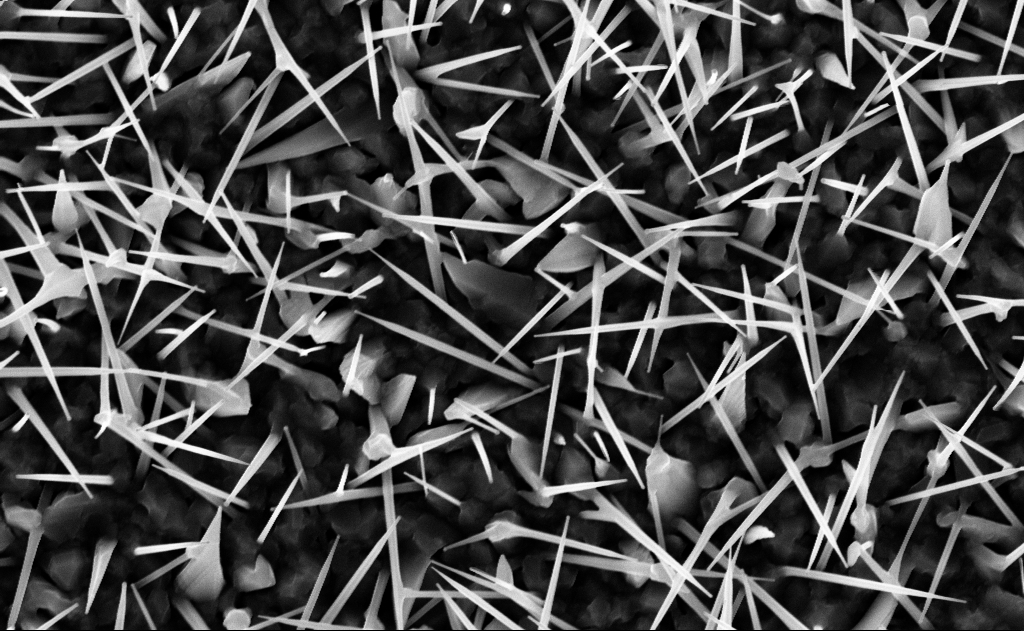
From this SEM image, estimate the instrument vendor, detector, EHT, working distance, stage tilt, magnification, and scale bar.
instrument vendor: Zeiss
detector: InLens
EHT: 10 kV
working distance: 9 mm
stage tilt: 0°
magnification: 40 K X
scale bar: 1000 nm